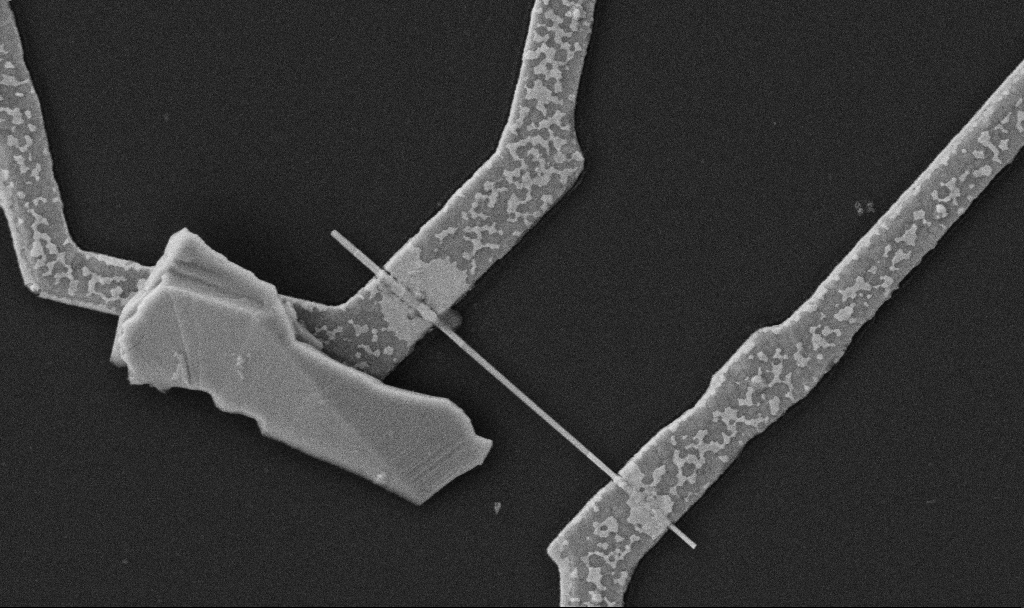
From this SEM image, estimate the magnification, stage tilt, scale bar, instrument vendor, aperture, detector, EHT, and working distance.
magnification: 30 K X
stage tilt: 0°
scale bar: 1000 nm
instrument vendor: Zeiss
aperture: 30 µm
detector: SE2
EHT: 5 kV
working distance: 10.7 mm